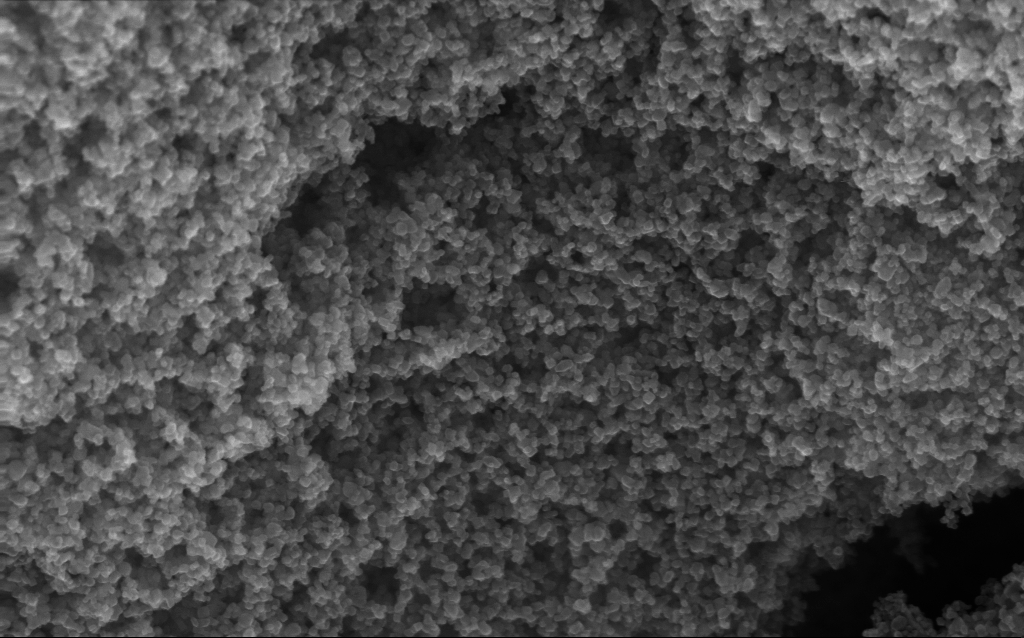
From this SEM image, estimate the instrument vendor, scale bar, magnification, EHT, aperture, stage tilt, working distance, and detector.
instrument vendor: Zeiss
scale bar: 100 nm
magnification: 130 K X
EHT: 10 kV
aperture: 30 µm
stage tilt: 0°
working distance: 2.7 mm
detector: InLens